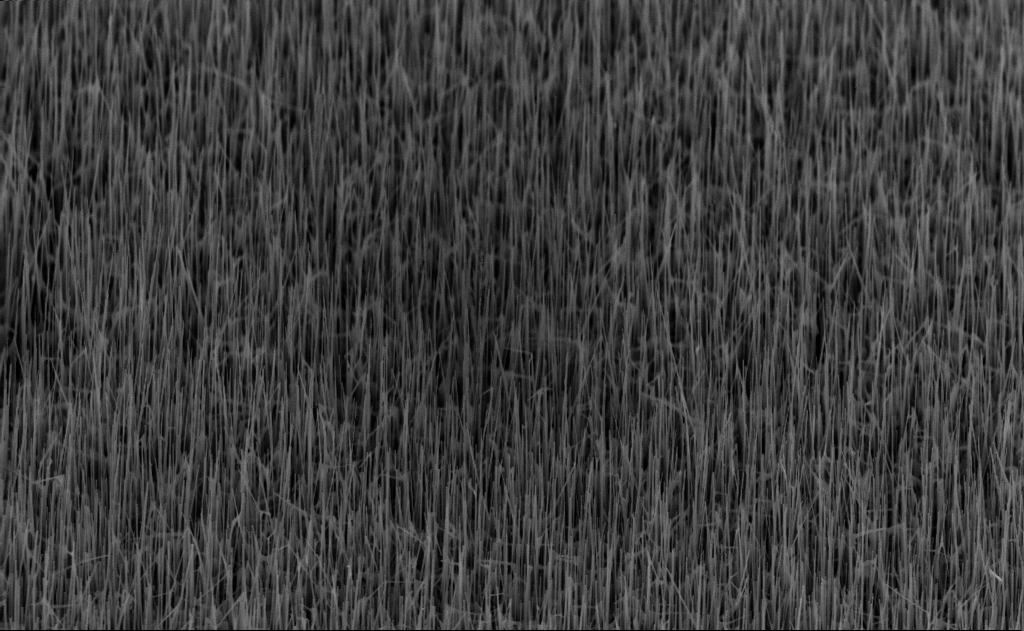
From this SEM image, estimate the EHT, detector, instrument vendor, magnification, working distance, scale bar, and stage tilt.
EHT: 10 kV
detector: InLens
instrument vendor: Zeiss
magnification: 20 K X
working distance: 6 mm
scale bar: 2000 nm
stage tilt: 45°